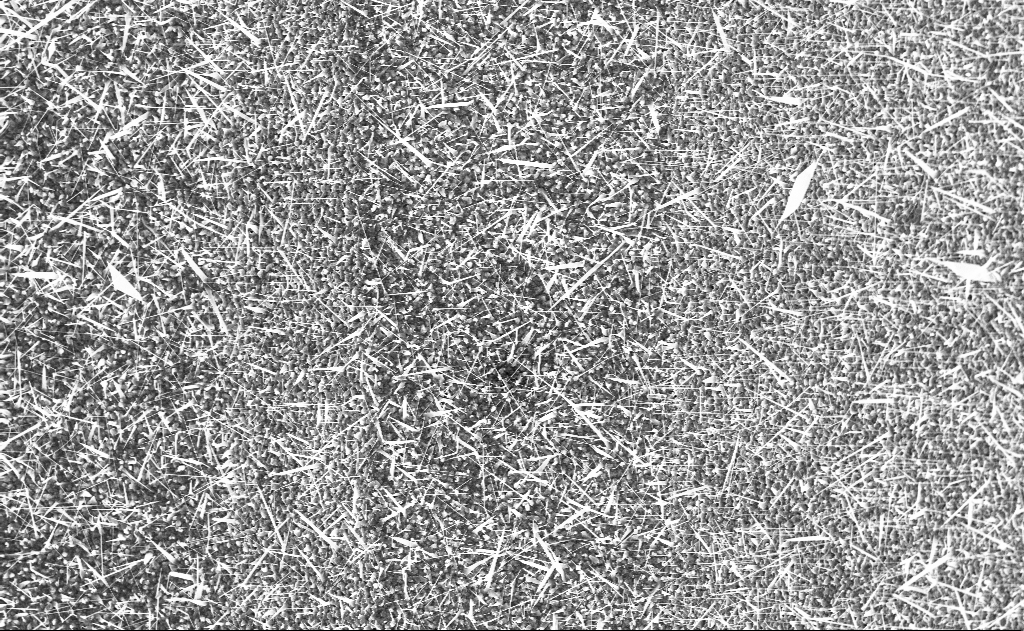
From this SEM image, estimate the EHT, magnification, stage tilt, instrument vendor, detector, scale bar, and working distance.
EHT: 10 kV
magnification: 5 K X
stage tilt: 0°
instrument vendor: Zeiss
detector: InLens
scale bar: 10000 nm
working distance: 9 mm